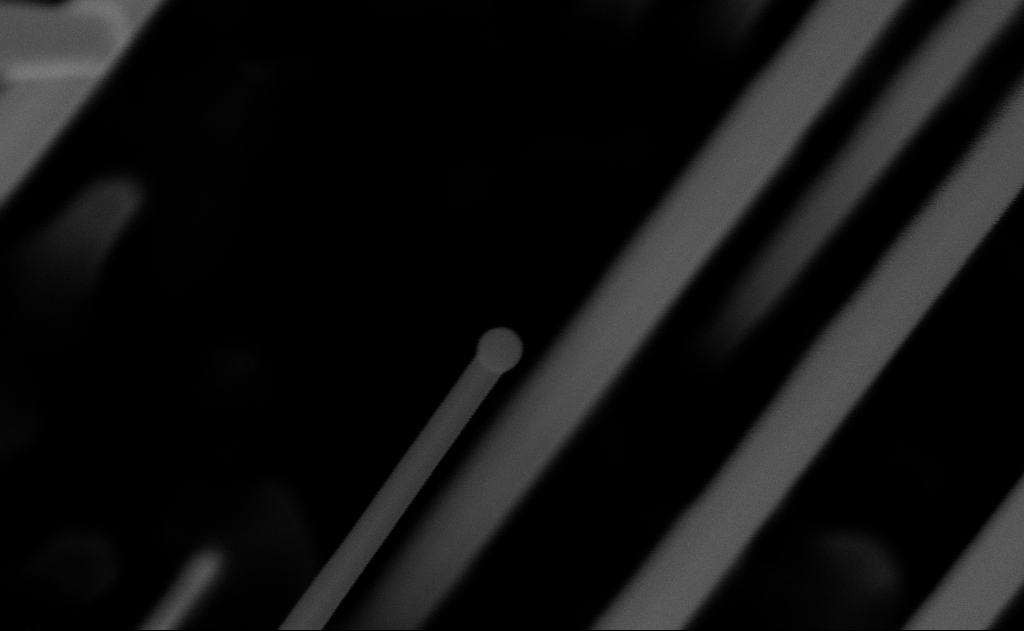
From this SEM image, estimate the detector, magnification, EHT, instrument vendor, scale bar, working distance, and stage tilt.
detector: InLens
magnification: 200 K X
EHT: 10 kV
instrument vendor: Zeiss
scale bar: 100 nm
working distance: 10 mm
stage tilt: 0°